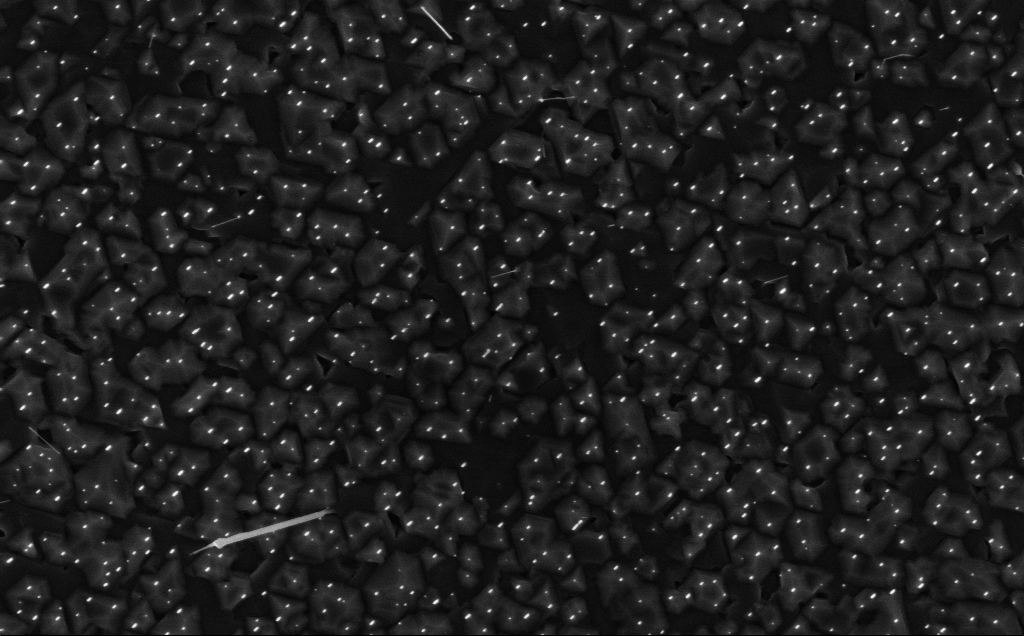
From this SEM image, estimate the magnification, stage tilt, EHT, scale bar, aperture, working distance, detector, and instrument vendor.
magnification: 20 K X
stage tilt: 0°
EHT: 7 kV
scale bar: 1000 nm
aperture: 30 µm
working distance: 4 mm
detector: InLens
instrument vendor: Zeiss